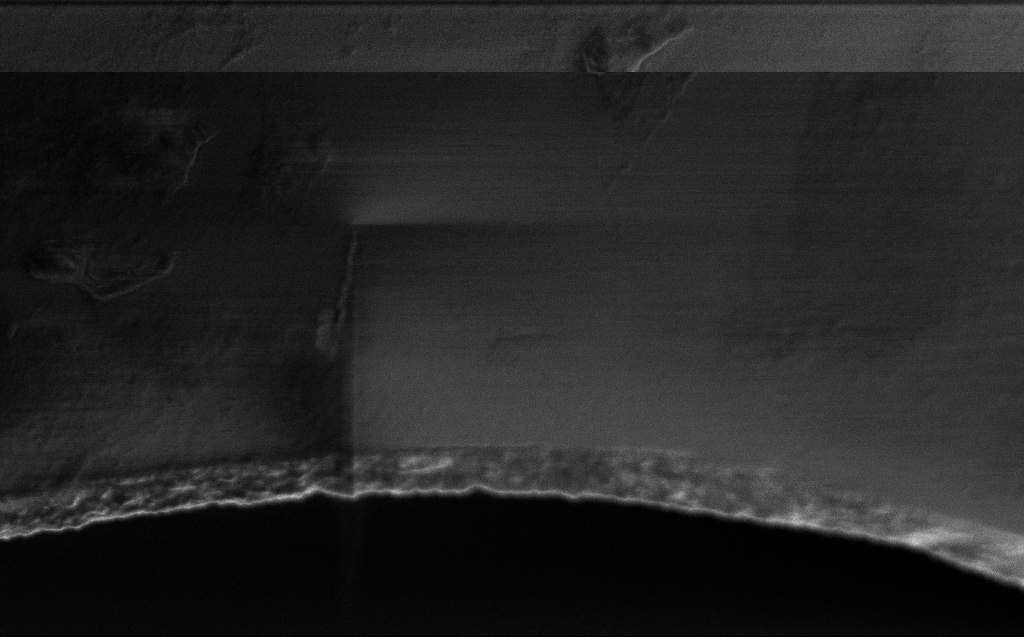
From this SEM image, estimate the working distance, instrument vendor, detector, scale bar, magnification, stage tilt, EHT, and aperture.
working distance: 3 mm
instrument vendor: Zeiss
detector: InLens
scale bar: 1000 nm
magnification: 40.54 K X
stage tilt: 45°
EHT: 1 kV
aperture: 30 µm